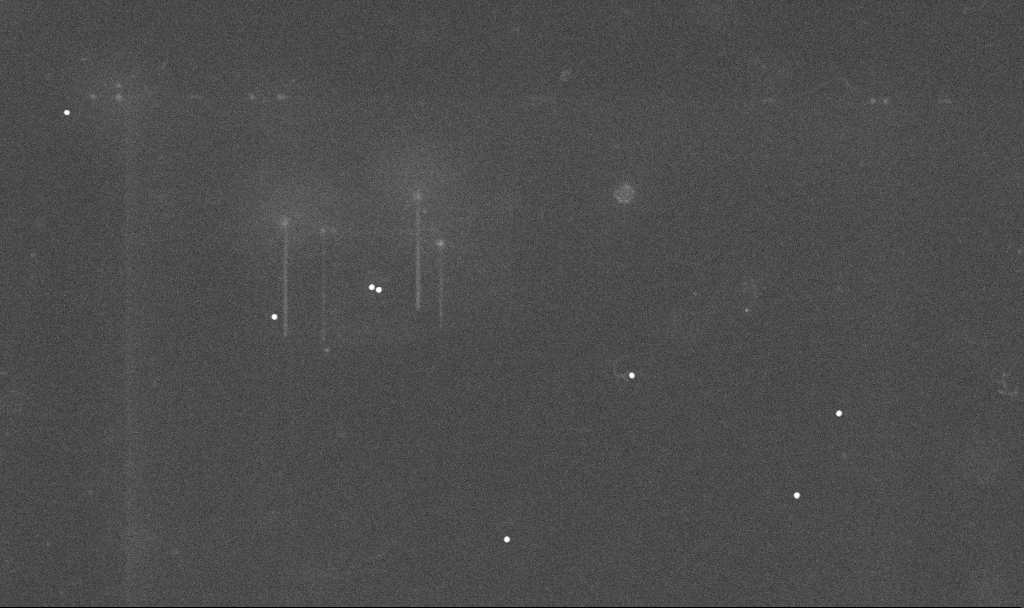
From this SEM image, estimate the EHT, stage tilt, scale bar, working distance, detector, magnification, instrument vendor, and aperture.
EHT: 10 kV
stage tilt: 0°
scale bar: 1000 nm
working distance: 3.4 mm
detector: InLens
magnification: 22.42 K X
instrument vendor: Zeiss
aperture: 30 µm